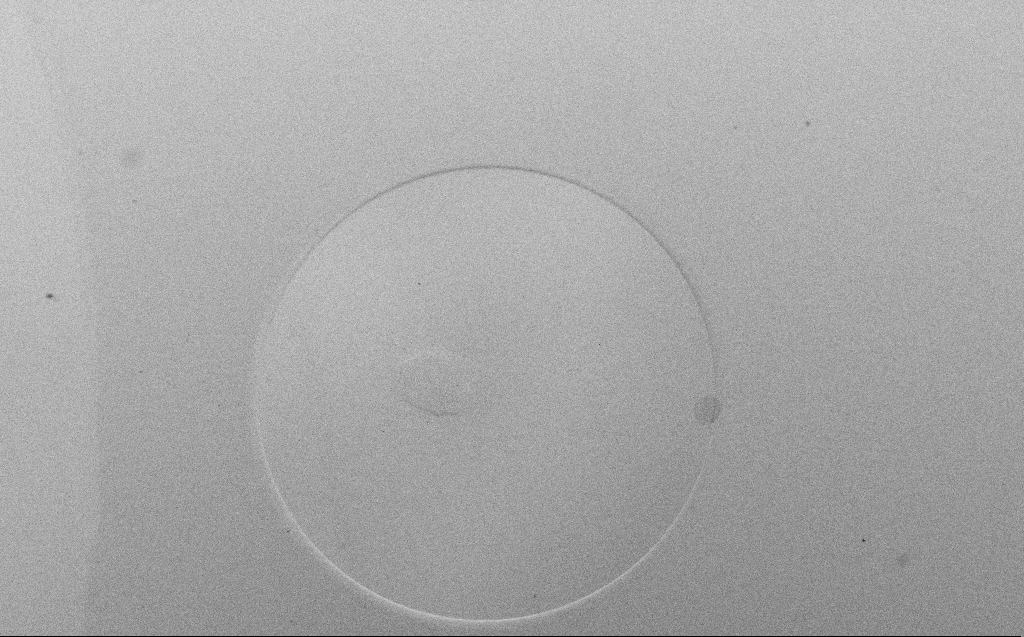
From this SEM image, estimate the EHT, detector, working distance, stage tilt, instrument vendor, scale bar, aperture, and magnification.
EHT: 5 kV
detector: SE2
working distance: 8 mm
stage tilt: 45°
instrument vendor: Zeiss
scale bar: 200000 nm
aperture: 30 µm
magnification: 0.087 K X